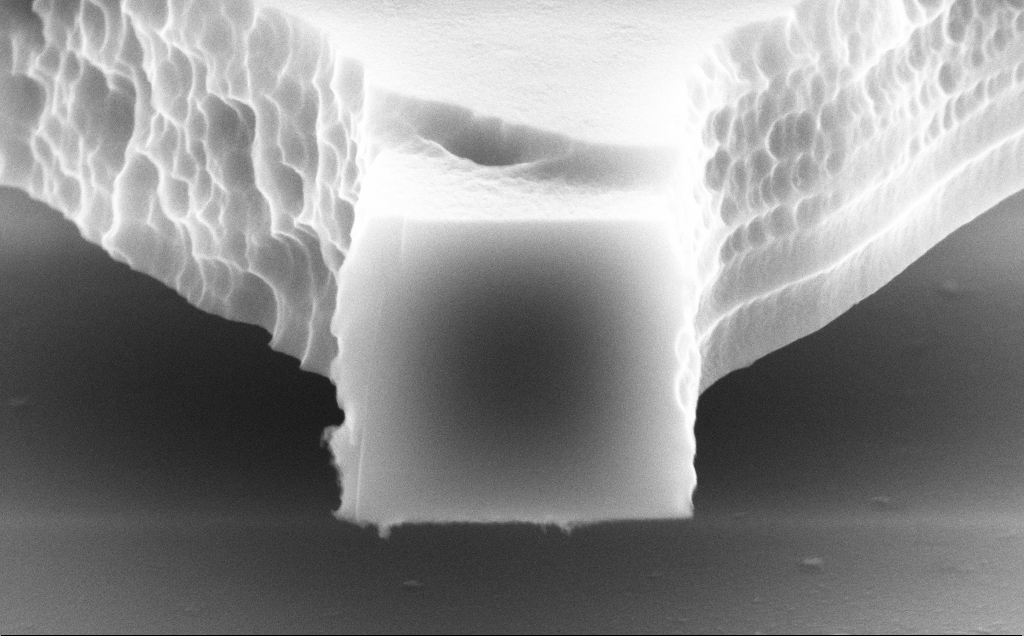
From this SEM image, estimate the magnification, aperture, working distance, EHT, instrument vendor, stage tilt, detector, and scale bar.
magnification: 53.67 K X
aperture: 30 µm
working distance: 9 mm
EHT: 10 kV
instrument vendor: Zeiss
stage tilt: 70°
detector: SE2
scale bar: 1000 nm